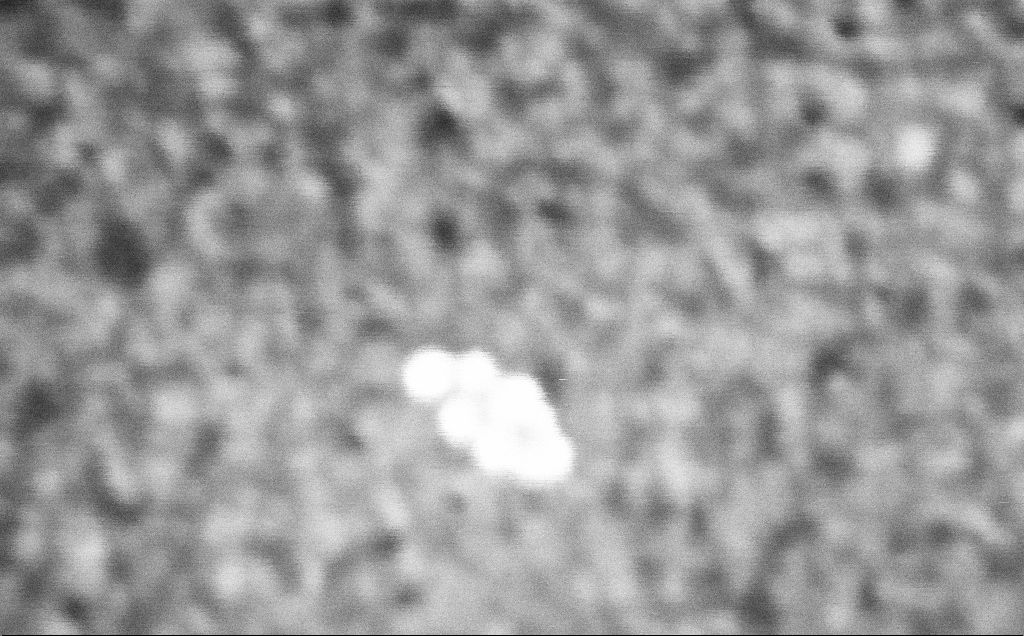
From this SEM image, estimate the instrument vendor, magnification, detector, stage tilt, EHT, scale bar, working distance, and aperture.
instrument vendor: Zeiss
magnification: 1124.39 K X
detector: InLens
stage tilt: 0°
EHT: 3 kV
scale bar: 20 nm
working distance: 3.2 mm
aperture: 30 µm